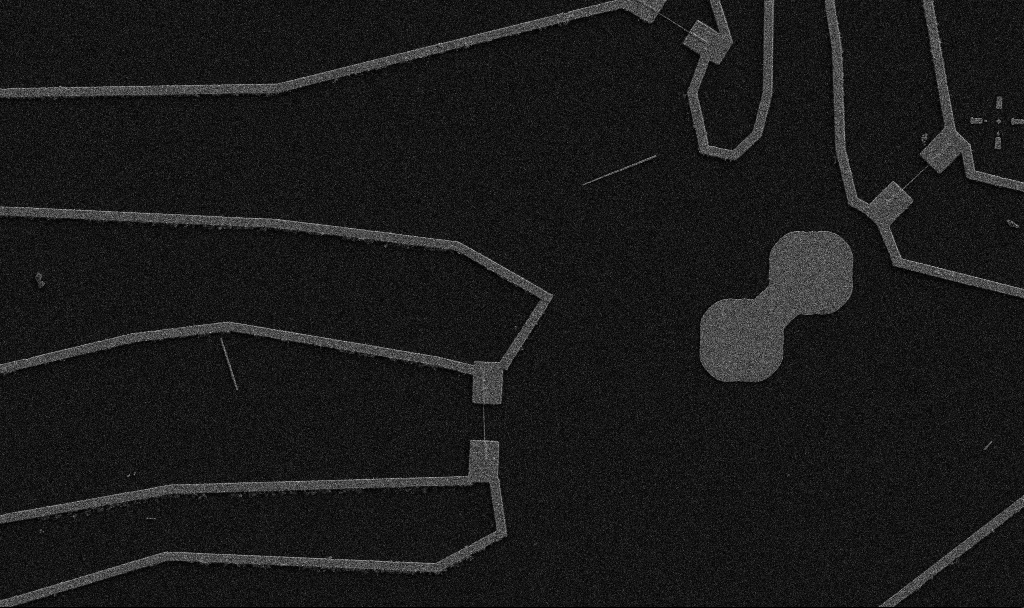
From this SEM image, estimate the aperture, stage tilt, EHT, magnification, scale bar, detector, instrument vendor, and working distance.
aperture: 30 µm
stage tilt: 0°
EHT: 5 kV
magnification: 5 K X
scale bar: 10000 nm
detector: SE2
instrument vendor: Zeiss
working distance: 10.7 mm